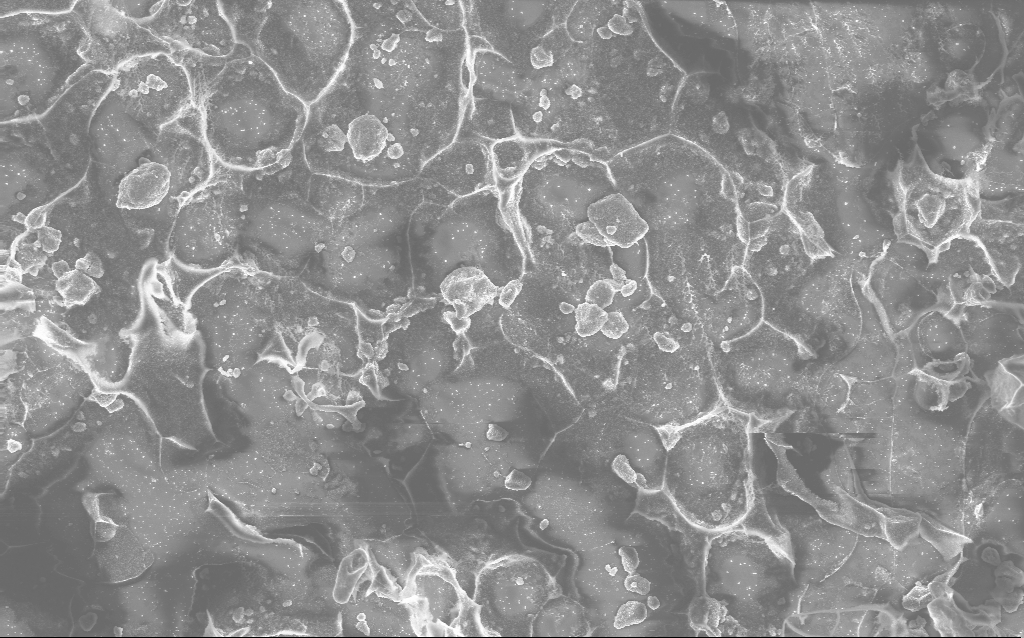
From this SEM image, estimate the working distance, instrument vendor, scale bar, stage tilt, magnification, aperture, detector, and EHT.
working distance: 2.8 mm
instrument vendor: Zeiss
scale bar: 10000 nm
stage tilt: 0°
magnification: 1.69 K X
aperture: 30 µm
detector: InLens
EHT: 10 kV